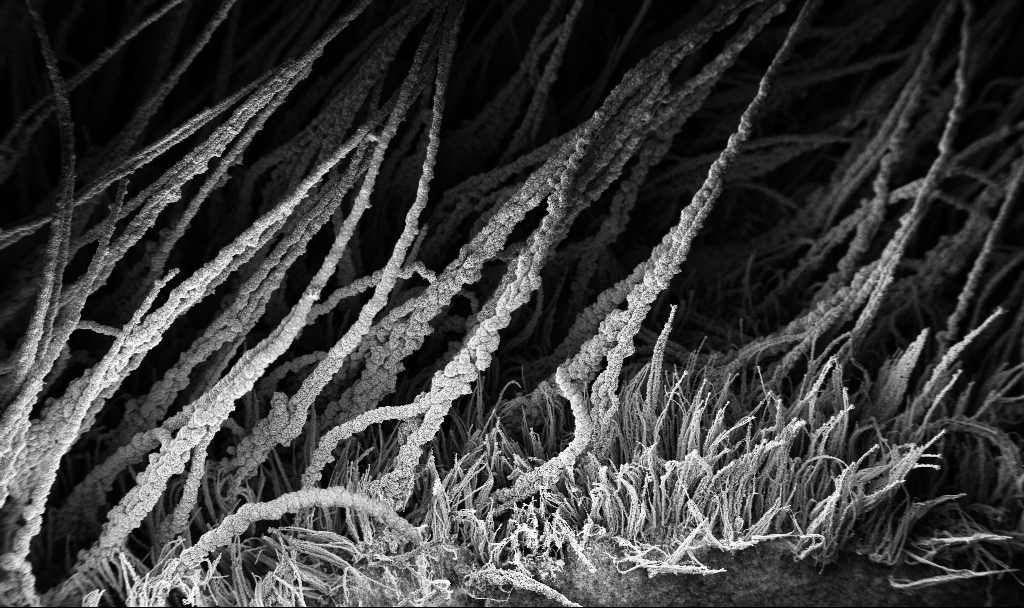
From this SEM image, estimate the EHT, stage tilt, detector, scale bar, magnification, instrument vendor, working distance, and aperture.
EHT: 3 kV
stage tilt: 37.7°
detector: InLens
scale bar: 100000 nm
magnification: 0.15 K X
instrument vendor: Zeiss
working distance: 7.5 mm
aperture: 30 µm